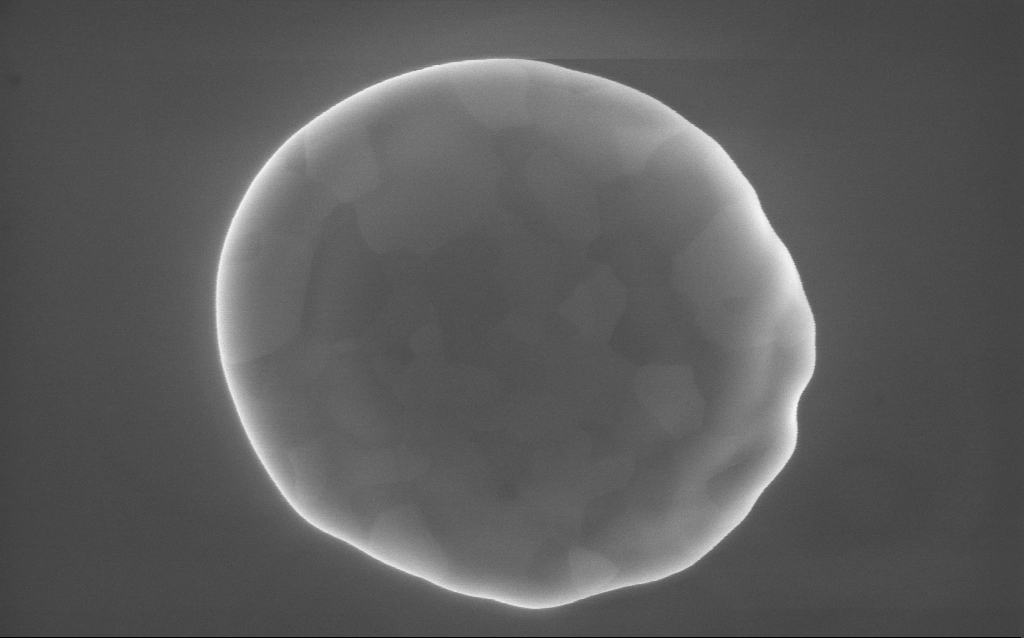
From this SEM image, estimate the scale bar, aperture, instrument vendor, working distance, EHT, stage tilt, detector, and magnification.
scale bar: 200 nm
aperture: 30 µm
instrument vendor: Zeiss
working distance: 2 mm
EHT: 10 kV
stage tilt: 0°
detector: InLens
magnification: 90 K X